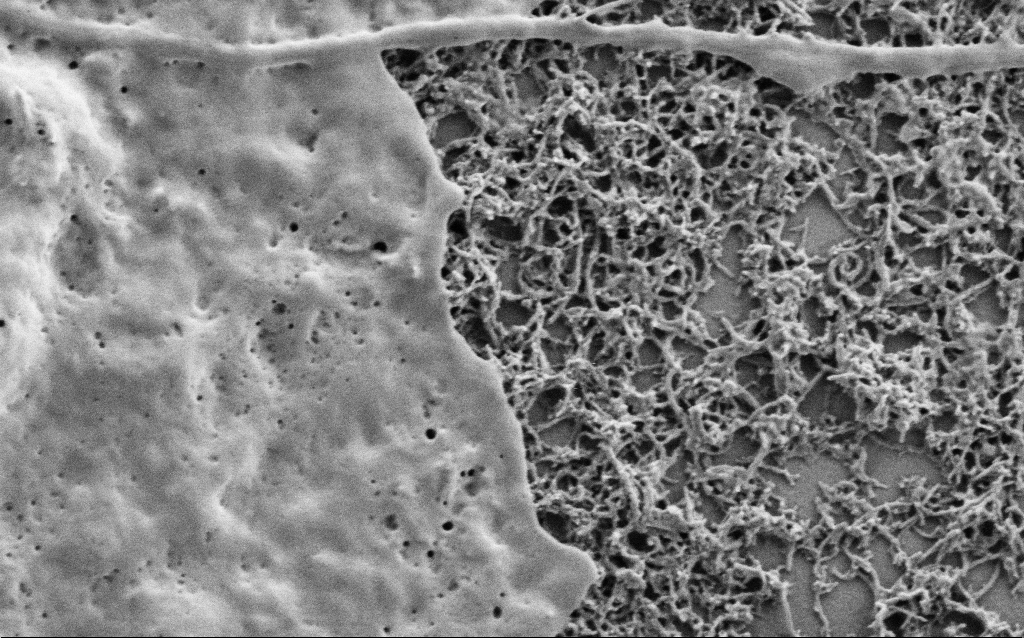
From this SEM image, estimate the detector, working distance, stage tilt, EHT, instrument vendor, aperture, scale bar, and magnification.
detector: SE2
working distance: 7 mm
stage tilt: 0°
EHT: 1 kV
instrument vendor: Zeiss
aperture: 30 µm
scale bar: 1000 nm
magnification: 50 K X